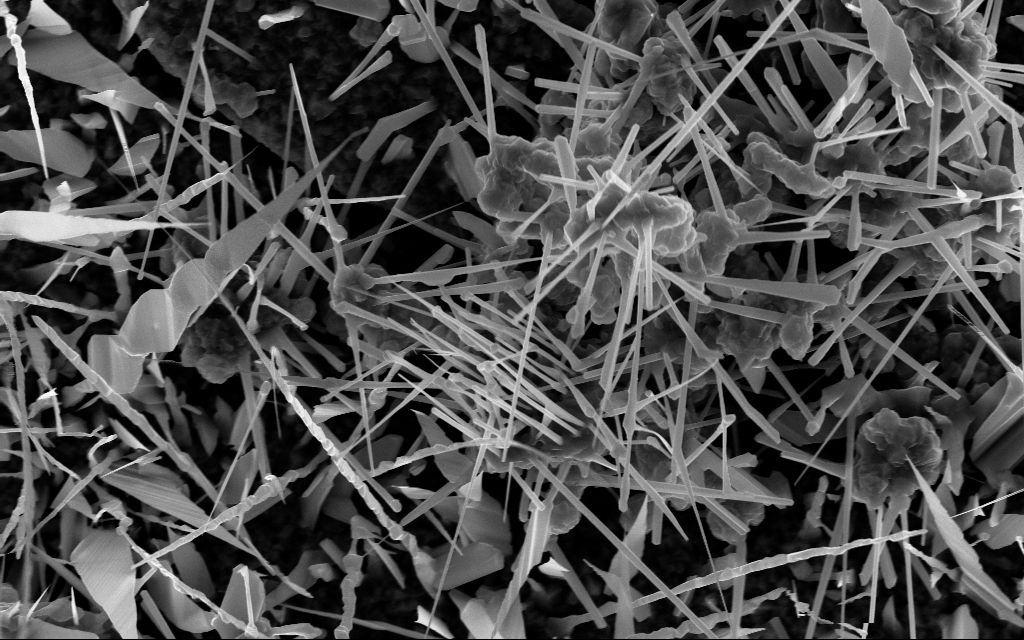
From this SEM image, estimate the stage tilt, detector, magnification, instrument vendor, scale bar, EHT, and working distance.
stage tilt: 0°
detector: InLens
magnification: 20 K X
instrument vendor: Zeiss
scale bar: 2000 nm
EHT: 10 kV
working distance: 6 mm